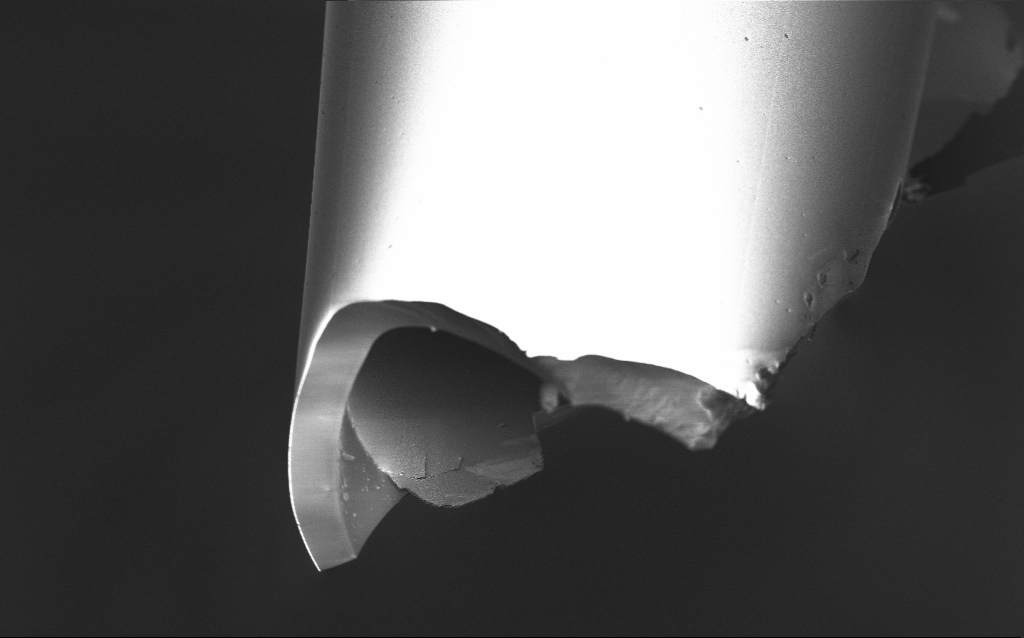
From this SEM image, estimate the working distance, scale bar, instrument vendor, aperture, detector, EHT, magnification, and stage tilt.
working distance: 5 mm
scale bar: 20000 nm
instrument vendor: Zeiss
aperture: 30 µm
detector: InLens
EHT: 1 kV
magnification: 2.5 K X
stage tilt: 45°